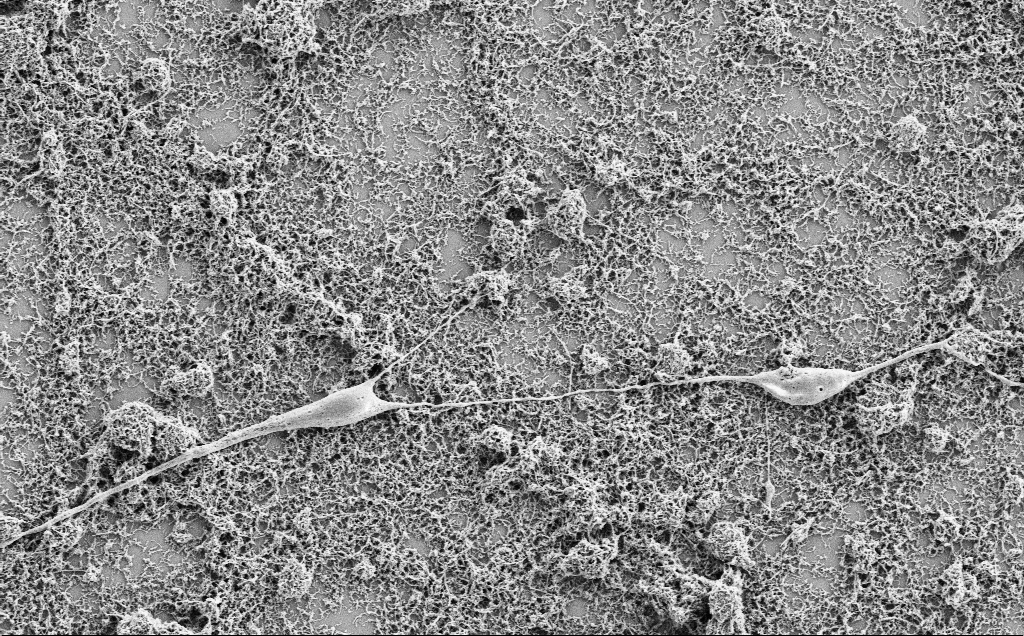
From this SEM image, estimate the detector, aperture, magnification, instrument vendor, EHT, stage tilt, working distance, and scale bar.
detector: SE2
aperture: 30 µm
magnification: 10 K X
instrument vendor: Zeiss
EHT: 2 kV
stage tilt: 0°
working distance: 7.1 mm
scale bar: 2000 nm